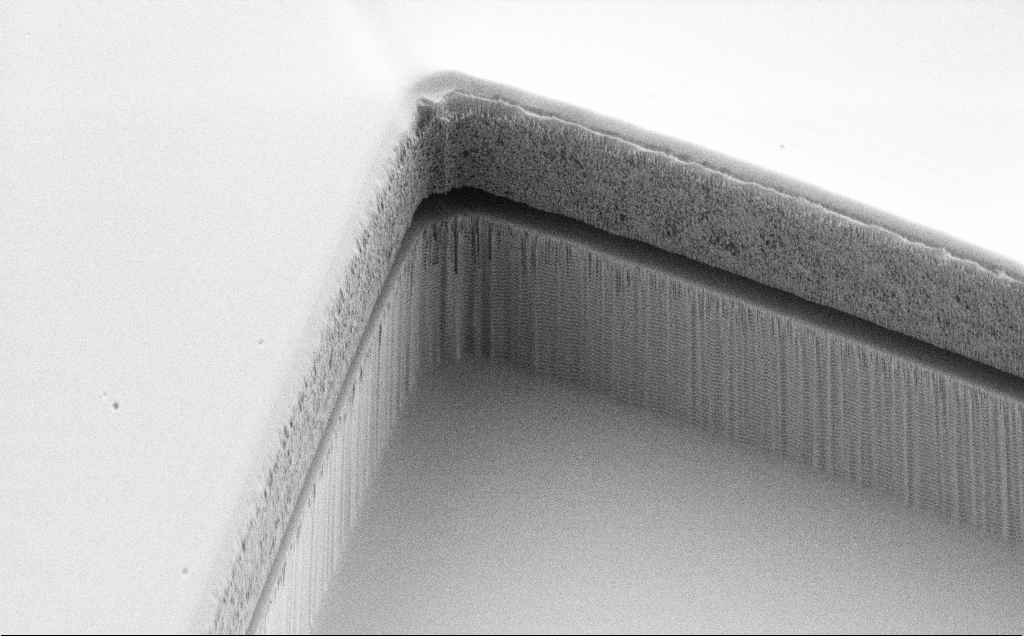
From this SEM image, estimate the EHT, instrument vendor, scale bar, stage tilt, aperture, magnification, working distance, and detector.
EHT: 1.2 kV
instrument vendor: Zeiss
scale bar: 10000 nm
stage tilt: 45°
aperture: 30 µm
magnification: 3.81 K X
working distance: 8 mm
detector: SE2